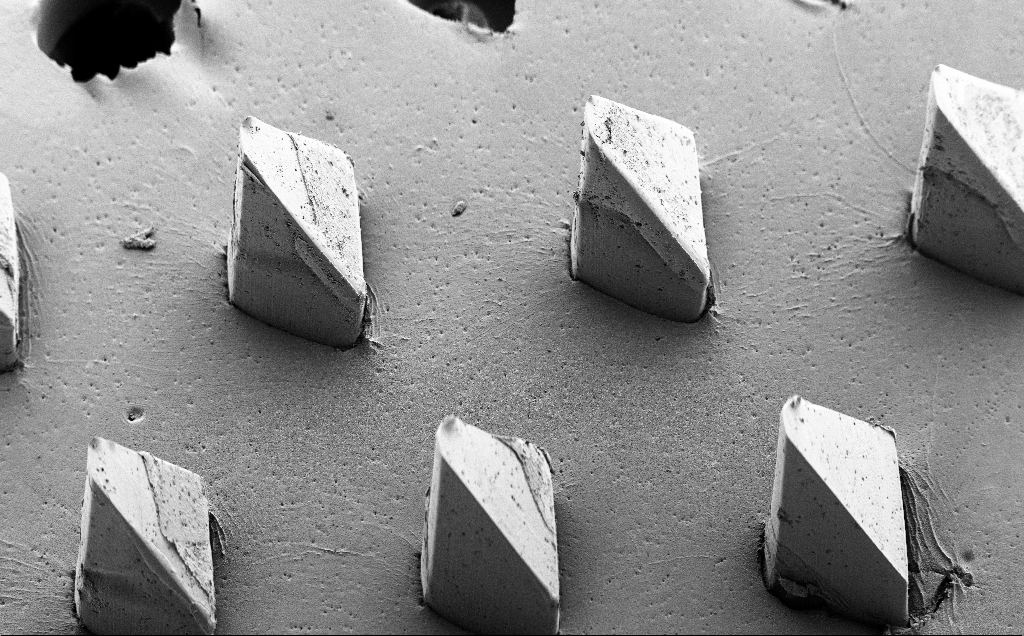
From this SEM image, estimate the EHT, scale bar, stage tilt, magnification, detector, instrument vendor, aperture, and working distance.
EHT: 5 kV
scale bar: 100000 nm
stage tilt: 40°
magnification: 0.135 K X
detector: SE2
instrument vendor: Zeiss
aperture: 30 µm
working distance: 9 mm